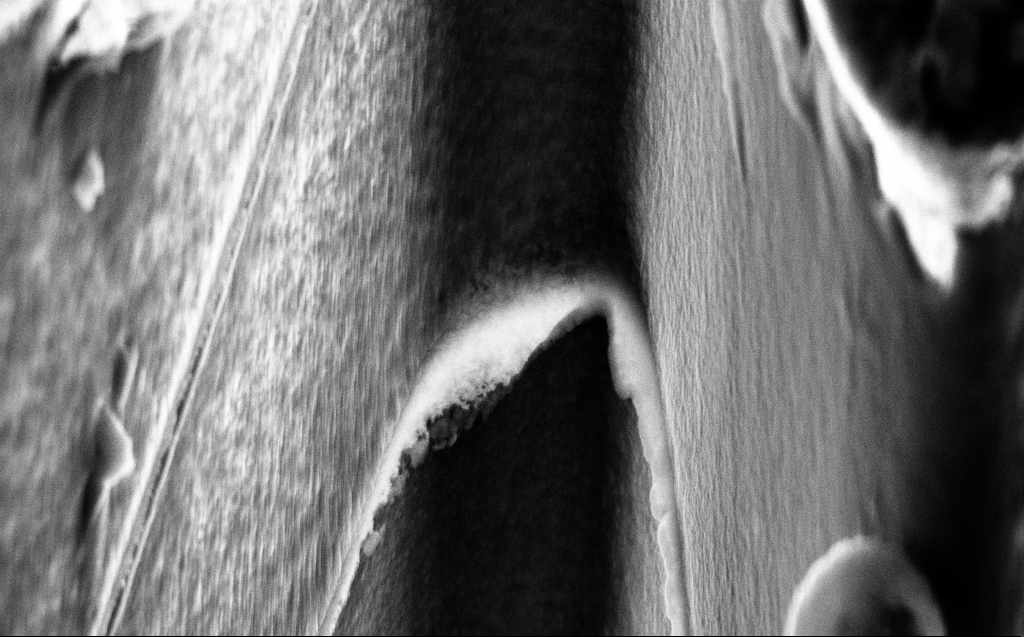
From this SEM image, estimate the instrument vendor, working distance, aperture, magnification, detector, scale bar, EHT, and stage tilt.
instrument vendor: Zeiss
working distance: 5 mm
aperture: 30 µm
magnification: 39.97 K X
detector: InLens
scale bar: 1000 nm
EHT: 10 kV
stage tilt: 45°